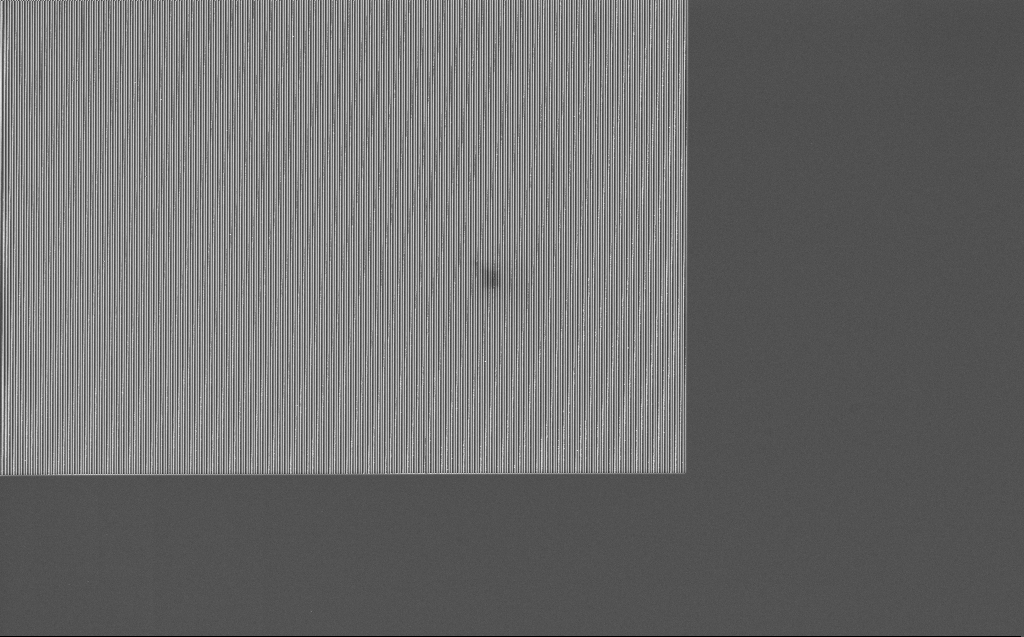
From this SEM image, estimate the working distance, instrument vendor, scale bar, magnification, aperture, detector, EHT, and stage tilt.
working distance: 7 mm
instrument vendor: Zeiss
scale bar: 10000 nm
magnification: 2.56 K X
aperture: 30 µm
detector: InLens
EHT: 5 kV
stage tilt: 0°